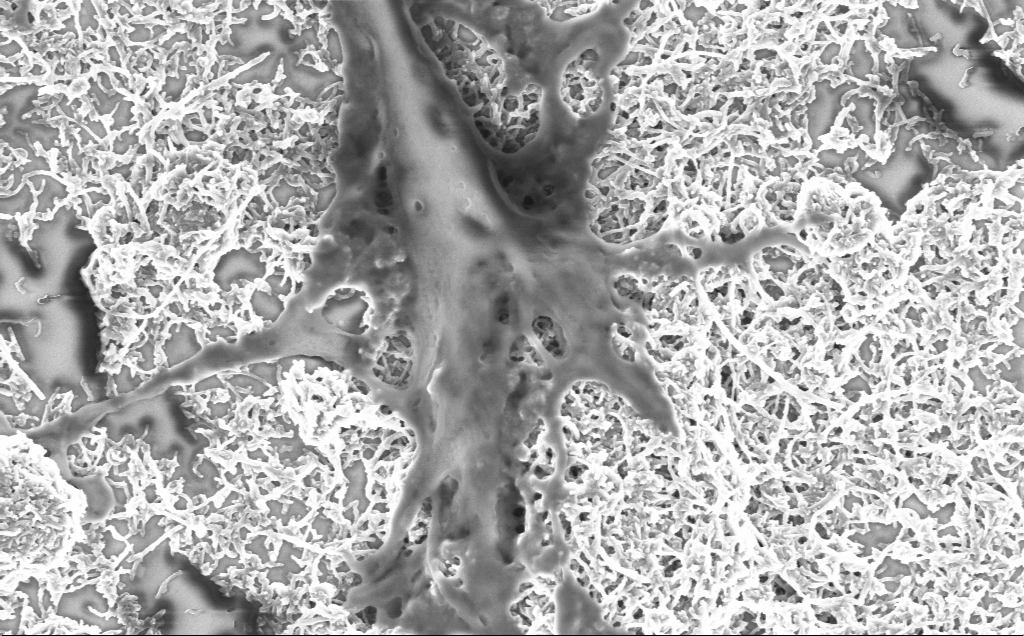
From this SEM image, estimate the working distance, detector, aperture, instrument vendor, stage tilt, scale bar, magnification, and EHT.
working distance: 7.1 mm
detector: InLens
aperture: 30 µm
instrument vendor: Zeiss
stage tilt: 0°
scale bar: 1000 nm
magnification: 40 K X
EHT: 2 kV